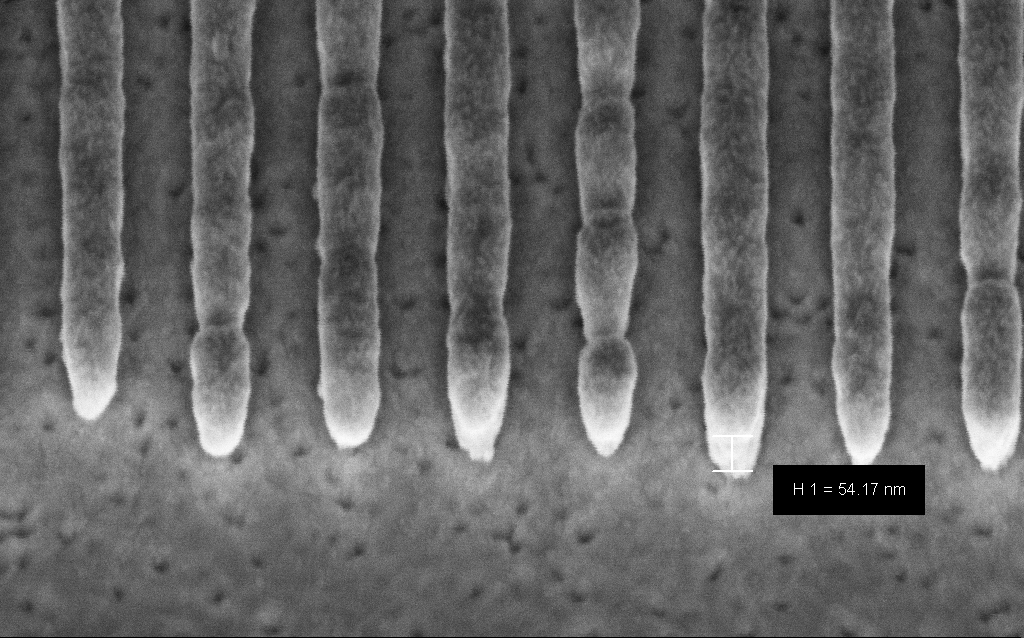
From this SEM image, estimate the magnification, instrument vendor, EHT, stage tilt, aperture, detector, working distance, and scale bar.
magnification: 237.25 K X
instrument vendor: Zeiss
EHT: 3 kV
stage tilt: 45°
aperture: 30 µm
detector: InLens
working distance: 6.6 mm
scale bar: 200 nm